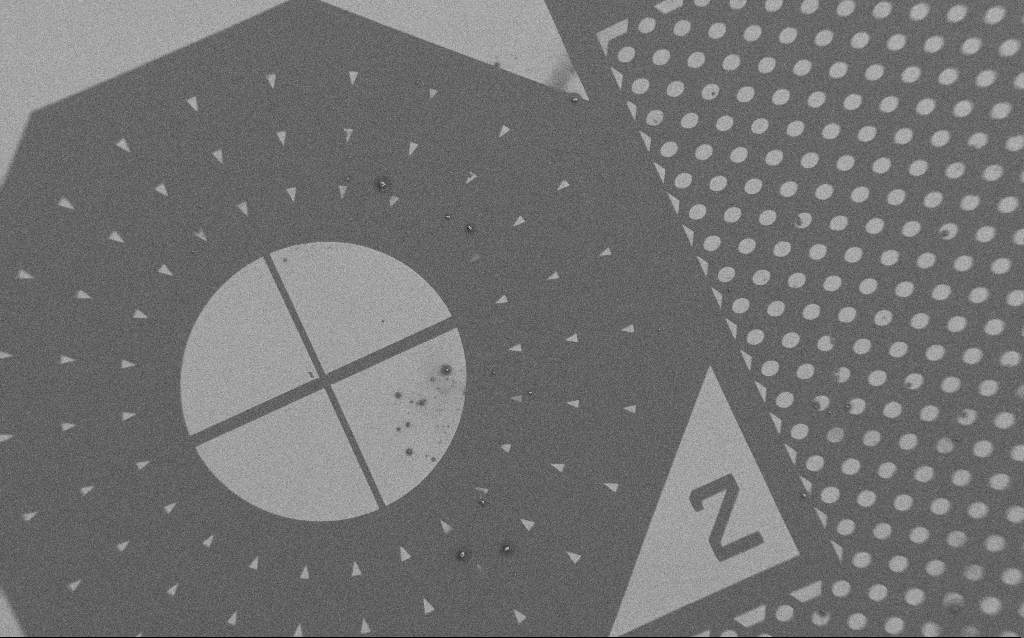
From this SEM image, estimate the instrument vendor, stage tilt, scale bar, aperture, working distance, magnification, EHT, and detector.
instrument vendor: Zeiss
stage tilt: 0°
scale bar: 100000 nm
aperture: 30 µm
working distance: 6 mm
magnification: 0.261 K X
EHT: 1.5 kV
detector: SE2